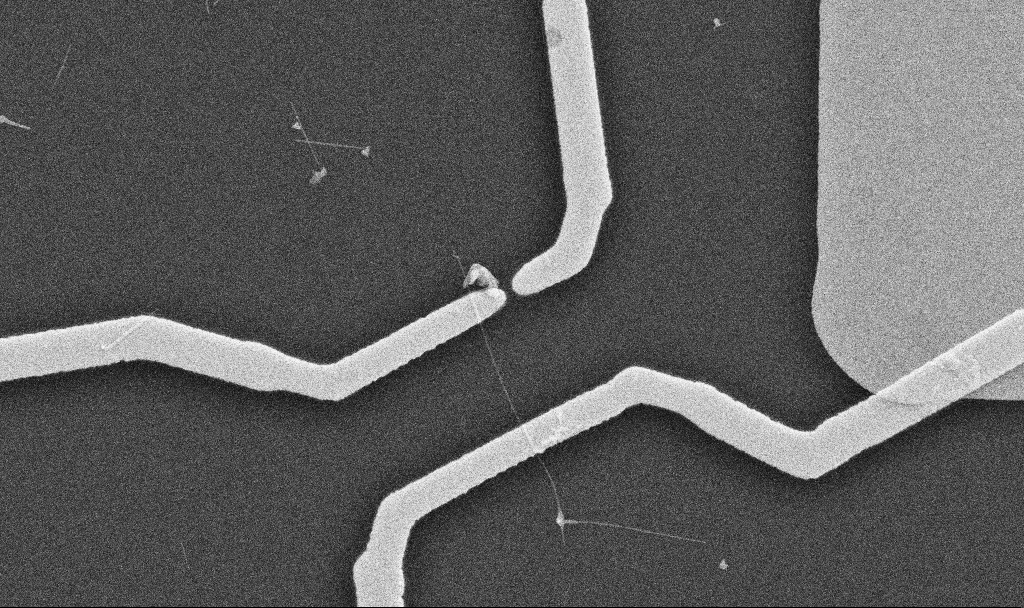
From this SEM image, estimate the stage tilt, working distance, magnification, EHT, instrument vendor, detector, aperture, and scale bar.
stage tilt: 0°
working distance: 10.7 mm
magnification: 20 K X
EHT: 10 kV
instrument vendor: Zeiss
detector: SE2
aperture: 30 µm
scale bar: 1000 nm